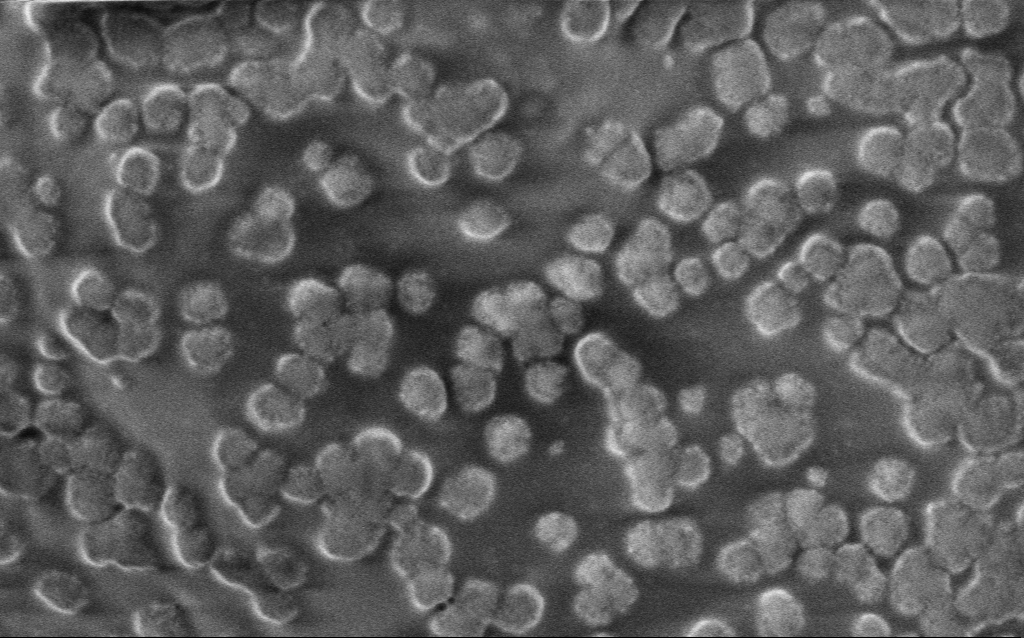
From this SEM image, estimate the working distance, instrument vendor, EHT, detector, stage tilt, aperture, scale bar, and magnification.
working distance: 4 mm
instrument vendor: Zeiss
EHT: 1 kV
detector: InLens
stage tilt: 0°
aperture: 30 µm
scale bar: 200 nm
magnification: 104.04 K X